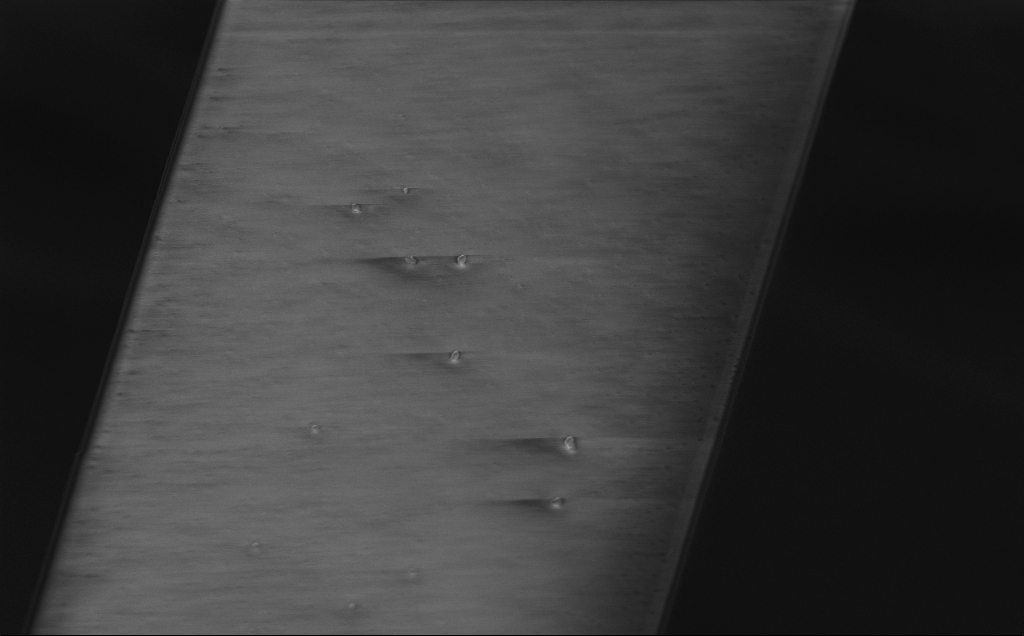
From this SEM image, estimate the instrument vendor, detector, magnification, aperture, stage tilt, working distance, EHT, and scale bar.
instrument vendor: Zeiss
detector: InLens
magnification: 6.06 K X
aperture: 30 µm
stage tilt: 0°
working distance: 7 mm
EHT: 5 kV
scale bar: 10000 nm